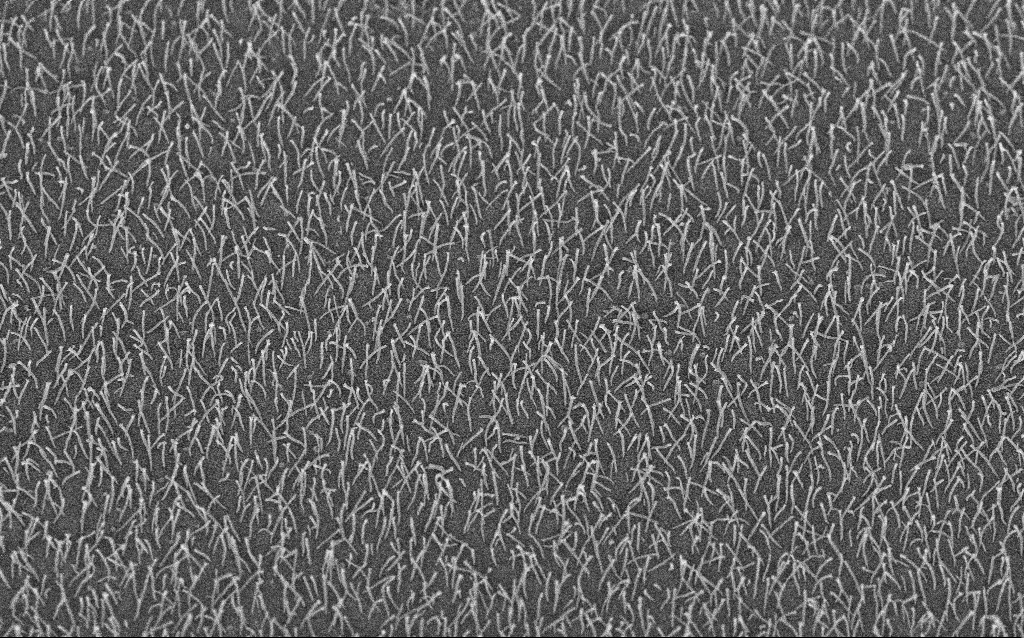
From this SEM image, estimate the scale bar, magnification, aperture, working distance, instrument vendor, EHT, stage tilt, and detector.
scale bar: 2000 nm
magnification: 10 K X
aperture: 30 µm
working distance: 8.3 mm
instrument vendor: Zeiss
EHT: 5 kV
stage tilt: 45°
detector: InLens